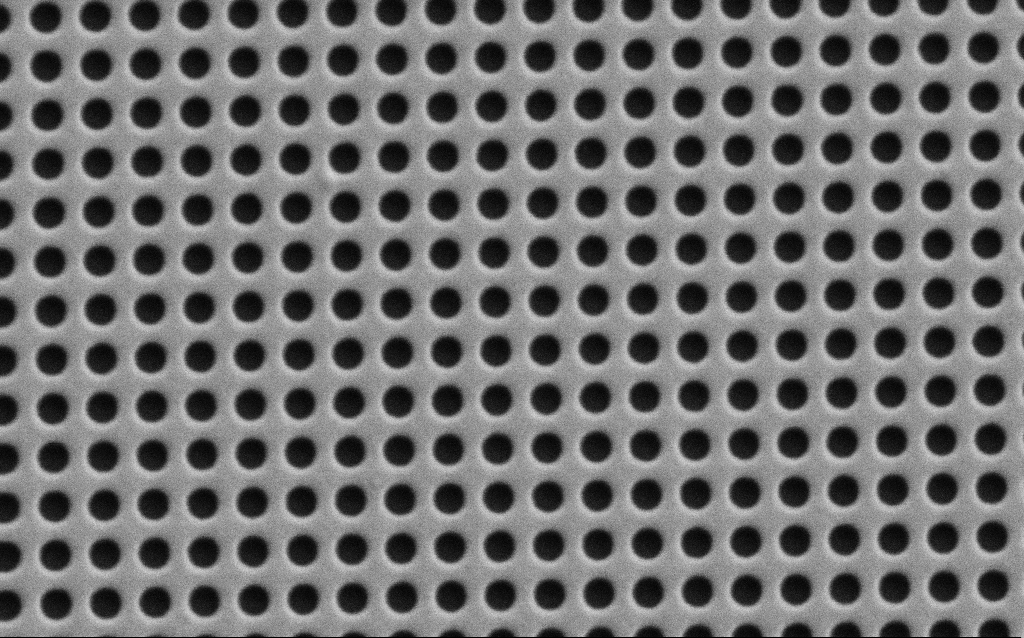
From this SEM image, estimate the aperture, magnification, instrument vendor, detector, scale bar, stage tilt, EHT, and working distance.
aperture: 30 µm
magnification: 59.88 K X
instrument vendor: Zeiss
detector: SE2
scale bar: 1000 nm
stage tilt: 0°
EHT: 1.5 kV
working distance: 5.8 mm